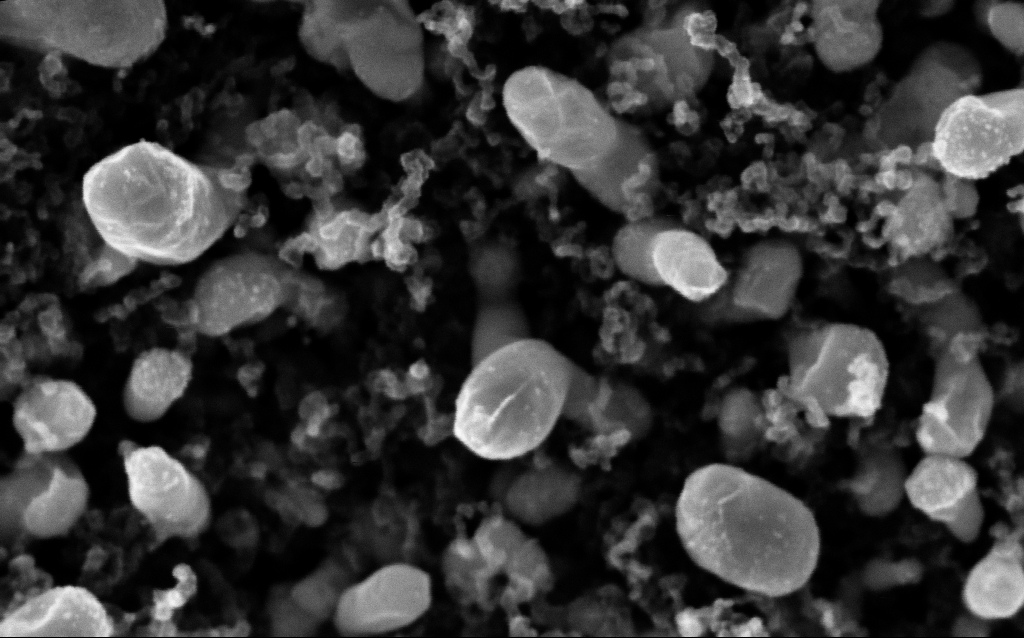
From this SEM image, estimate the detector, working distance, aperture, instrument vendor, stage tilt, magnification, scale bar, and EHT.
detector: InLens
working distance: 2.3 mm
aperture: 30 µm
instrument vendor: Zeiss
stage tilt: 0°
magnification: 200 K X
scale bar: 100 nm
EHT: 5 kV